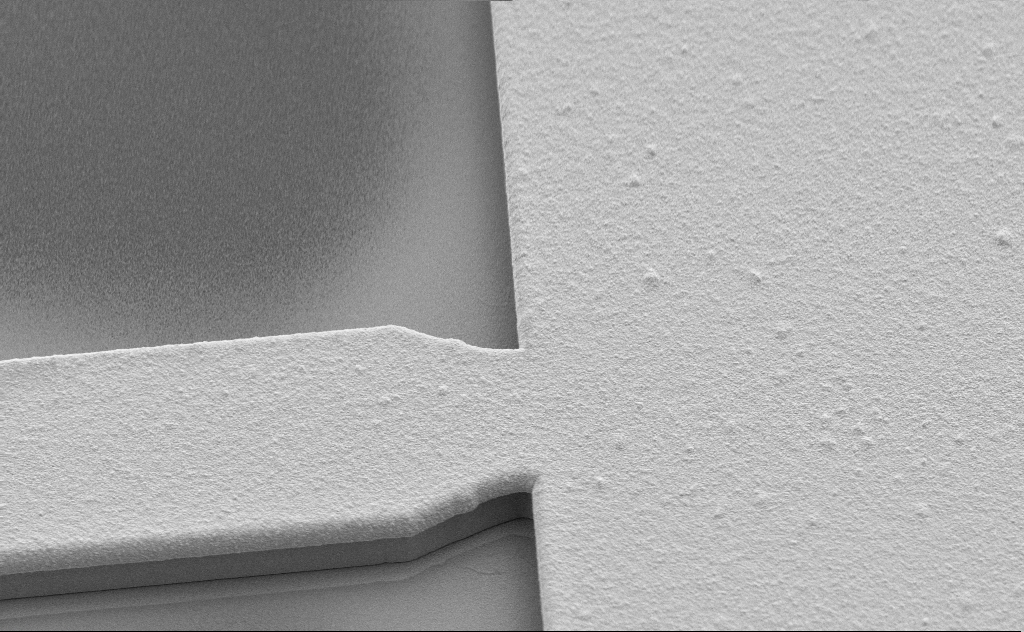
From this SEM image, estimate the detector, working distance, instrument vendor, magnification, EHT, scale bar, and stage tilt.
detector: SE2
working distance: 8 mm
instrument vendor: Zeiss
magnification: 6.75 K X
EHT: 5 kV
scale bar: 10000 nm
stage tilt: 45°